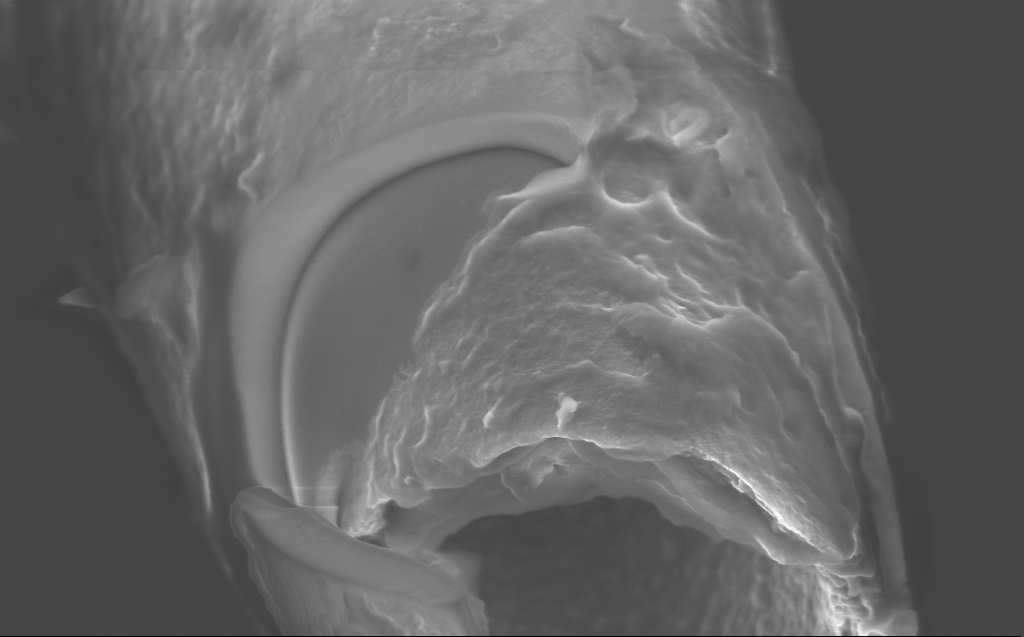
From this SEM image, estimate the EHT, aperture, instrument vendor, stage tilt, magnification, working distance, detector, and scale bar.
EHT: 3 kV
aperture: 30 µm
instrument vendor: Zeiss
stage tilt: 45°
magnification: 63.75 K X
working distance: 4 mm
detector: InLens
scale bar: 1000 nm